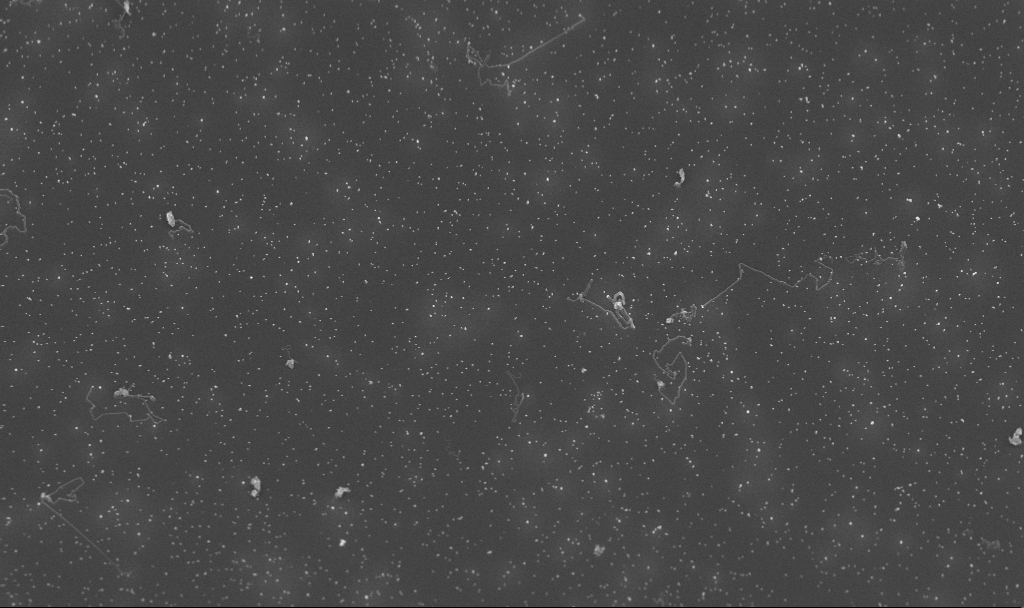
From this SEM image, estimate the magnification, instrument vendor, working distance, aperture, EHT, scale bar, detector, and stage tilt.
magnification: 19.18 K X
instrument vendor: Zeiss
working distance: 4.9 mm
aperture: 30 µm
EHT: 10 kV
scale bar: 2000 nm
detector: InLens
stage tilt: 45°